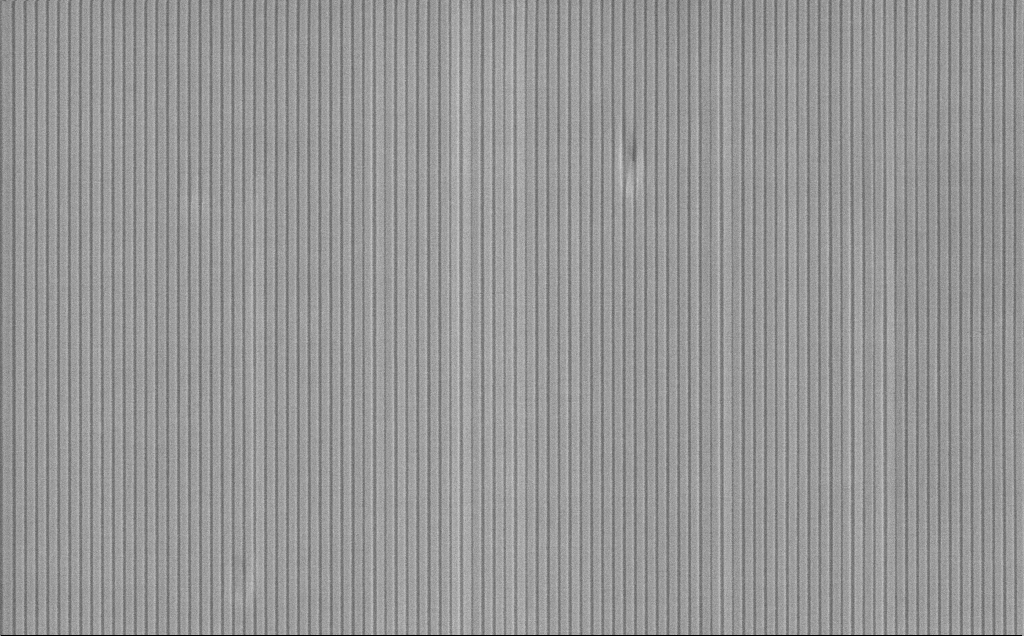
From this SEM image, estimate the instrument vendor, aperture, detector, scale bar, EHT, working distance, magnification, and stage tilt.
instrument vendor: Zeiss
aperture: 30 µm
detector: SE2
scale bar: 1000 nm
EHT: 10 kV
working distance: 10 mm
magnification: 22.4 K X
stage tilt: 45°